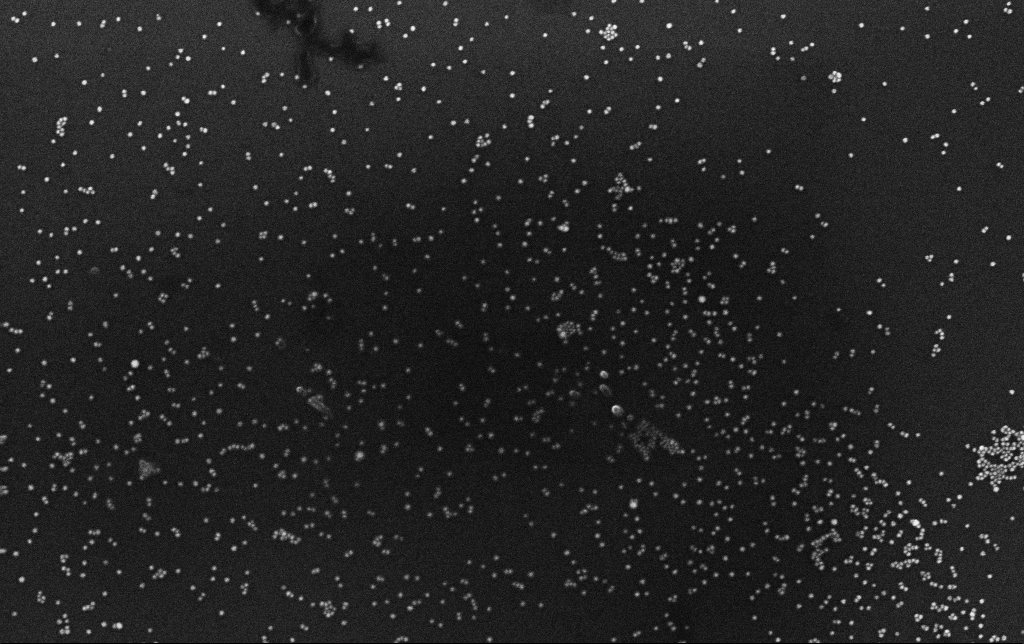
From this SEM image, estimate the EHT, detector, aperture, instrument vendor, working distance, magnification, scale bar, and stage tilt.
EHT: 10 kV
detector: InLens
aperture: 30 µm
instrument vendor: Zeiss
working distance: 3.1 mm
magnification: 100 K X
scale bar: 200 nm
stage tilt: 0°